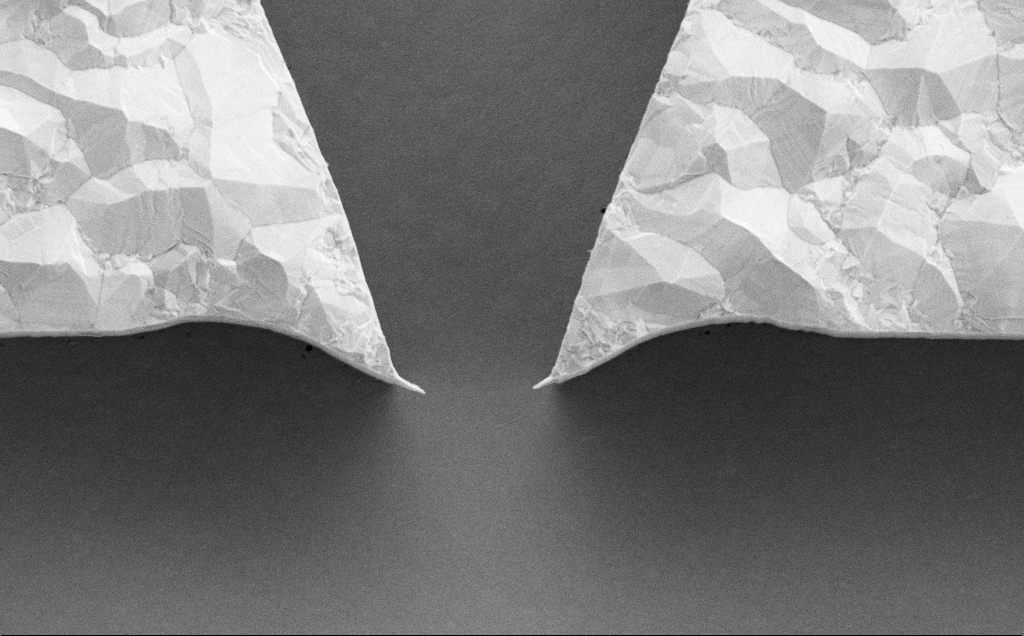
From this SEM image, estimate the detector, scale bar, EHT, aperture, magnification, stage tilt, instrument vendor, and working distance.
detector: SE2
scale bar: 2000 nm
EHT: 5 kV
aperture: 30 µm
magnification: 17.29 K X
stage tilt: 0°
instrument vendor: Zeiss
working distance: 13 mm